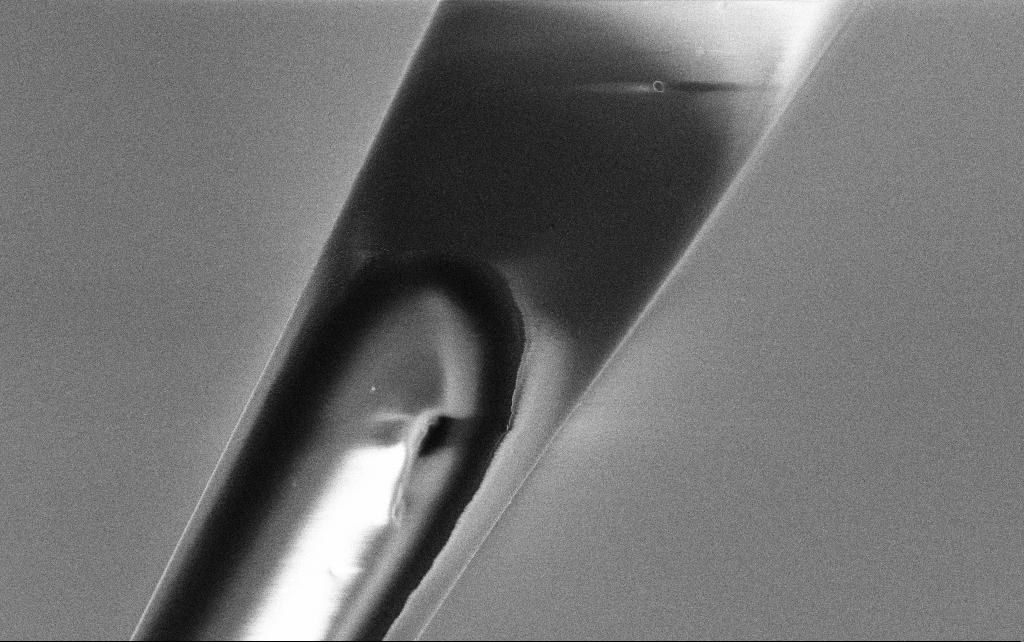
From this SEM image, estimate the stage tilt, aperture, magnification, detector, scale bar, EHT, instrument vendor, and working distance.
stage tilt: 45°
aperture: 30 µm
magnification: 10 K X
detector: InLens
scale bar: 2000 nm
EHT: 3 kV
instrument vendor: Zeiss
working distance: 7.6 mm